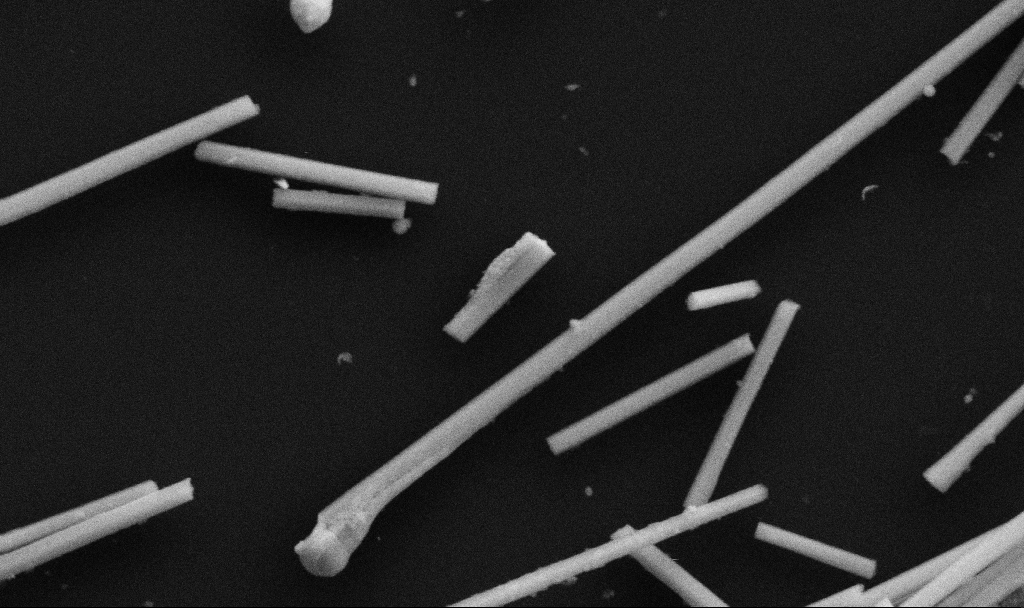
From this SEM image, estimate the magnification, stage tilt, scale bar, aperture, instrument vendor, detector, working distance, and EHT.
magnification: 84.27 K X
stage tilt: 0°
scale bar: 200 nm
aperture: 30 µm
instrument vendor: Zeiss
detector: SE2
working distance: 6.7 mm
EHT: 5 kV